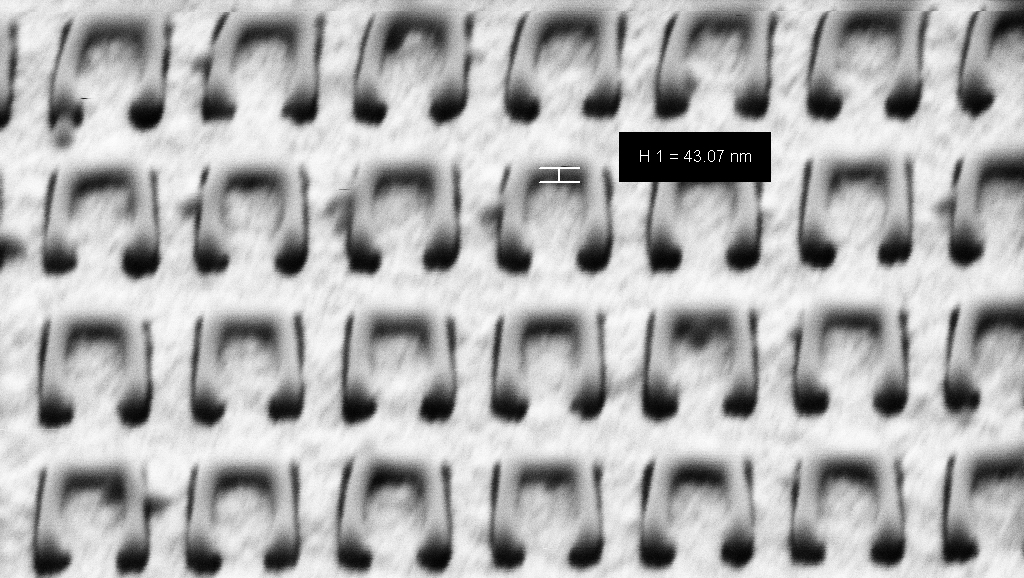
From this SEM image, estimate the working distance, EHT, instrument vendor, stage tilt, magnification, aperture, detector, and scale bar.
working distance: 7.6 mm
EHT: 1.5 kV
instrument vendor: Zeiss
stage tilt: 45°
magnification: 119.36 K X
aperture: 30 µm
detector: SE2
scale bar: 200 nm